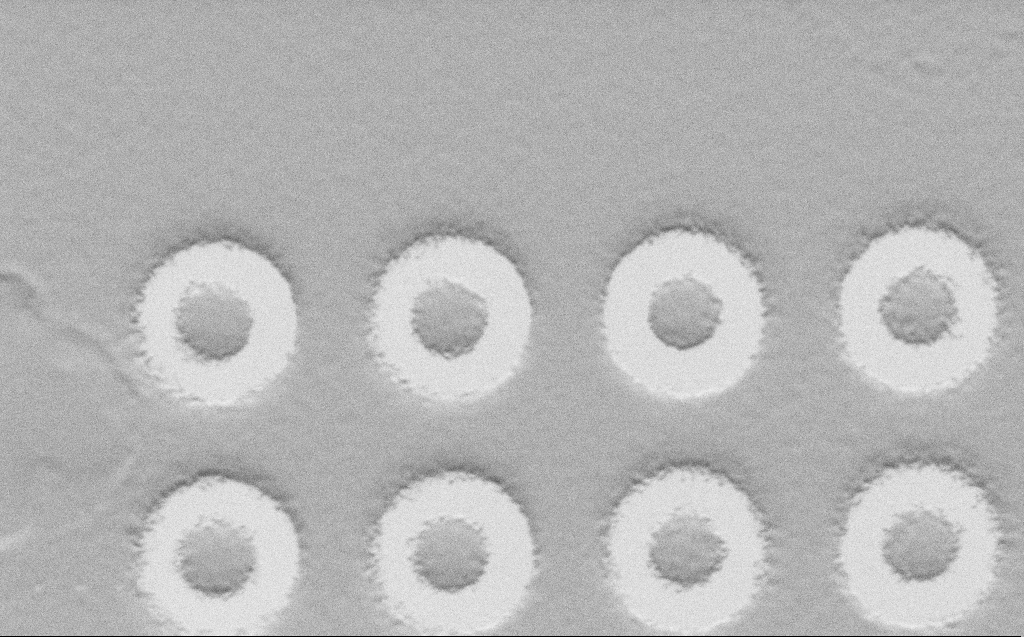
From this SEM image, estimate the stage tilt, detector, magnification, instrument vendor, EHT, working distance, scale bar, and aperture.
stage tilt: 45°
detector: SE2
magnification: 8.73 K X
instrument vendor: Zeiss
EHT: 1 kV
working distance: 5 mm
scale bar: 2000 nm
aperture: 30 µm